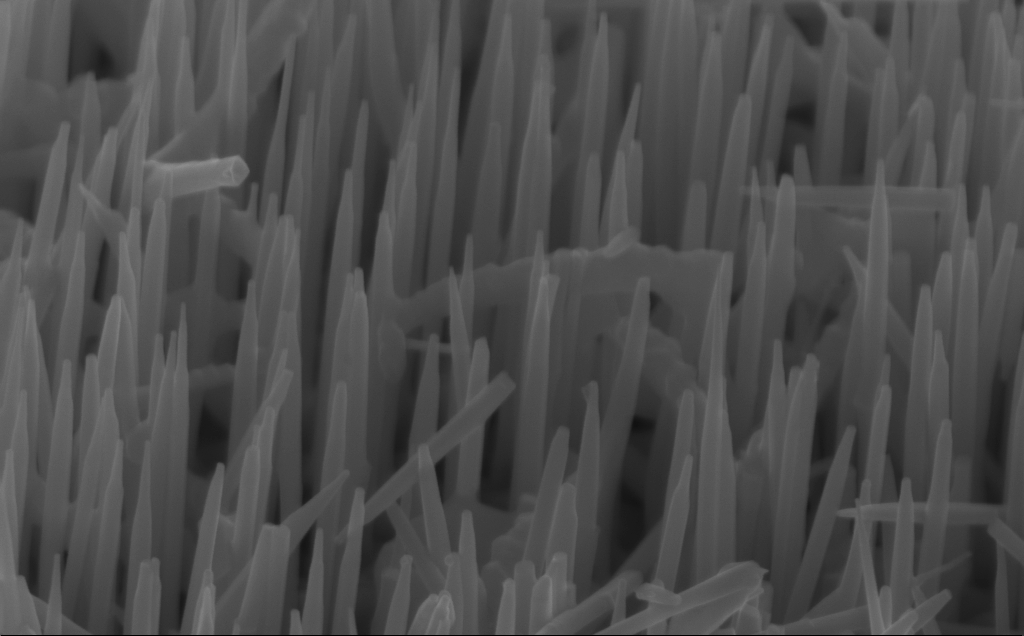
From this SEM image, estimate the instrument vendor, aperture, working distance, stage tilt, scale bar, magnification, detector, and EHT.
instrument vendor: Zeiss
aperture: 30 µm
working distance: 5 mm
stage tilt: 45°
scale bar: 200 nm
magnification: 80 K X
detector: InLens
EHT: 12 kV